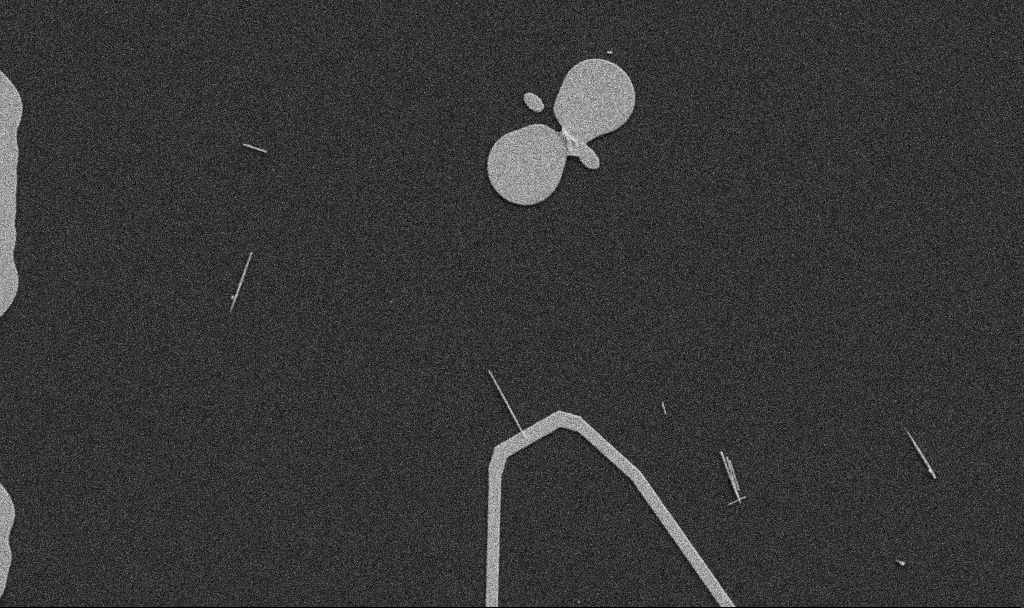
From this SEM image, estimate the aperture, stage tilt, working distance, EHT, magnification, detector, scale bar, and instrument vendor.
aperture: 30 µm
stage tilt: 0°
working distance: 10.7 mm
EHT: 5 kV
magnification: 5 K X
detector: SE2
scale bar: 10000 nm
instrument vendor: Zeiss